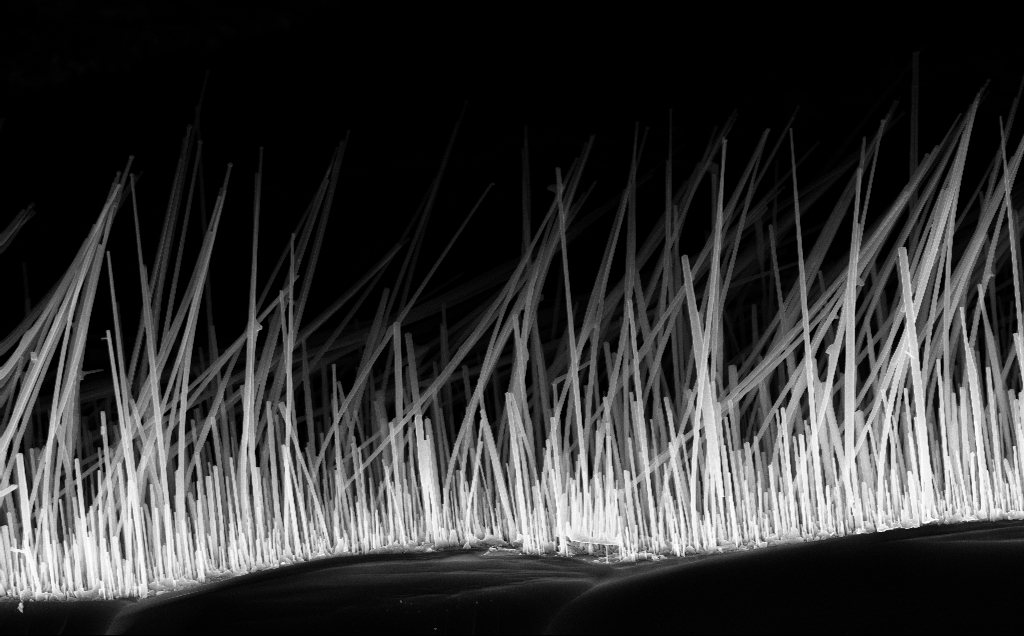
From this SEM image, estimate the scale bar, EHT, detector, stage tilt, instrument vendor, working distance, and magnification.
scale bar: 1000 nm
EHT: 10 kV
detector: InLens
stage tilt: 30°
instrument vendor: Zeiss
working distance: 7 mm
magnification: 17.86 K X